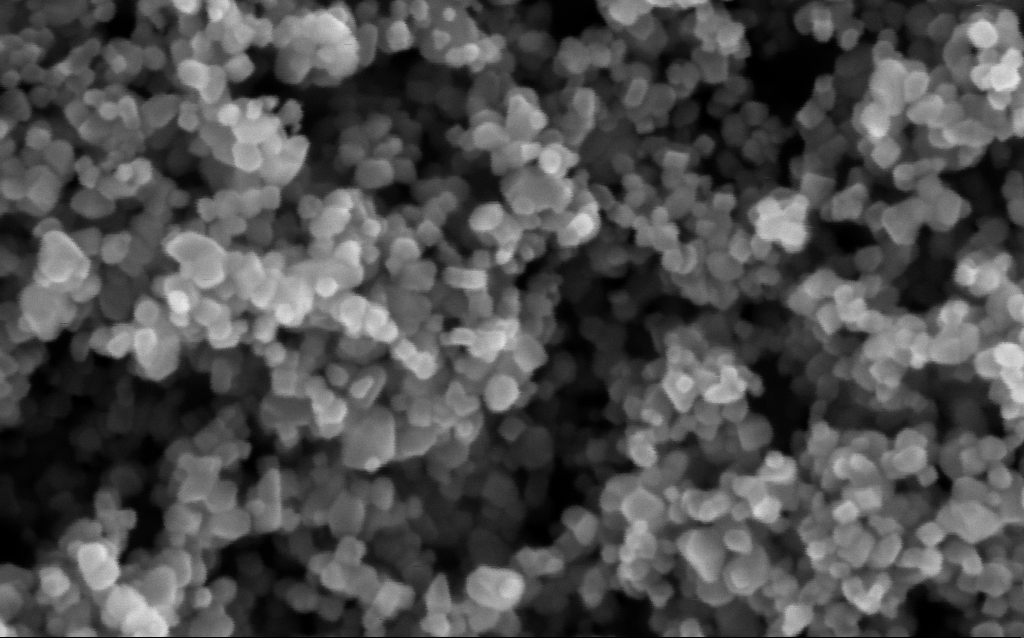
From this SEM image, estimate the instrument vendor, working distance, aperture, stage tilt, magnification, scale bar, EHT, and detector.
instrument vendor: Zeiss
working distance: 5.3 mm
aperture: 30 µm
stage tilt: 0°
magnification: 416 K X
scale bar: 100 nm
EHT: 5 kV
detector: InLens